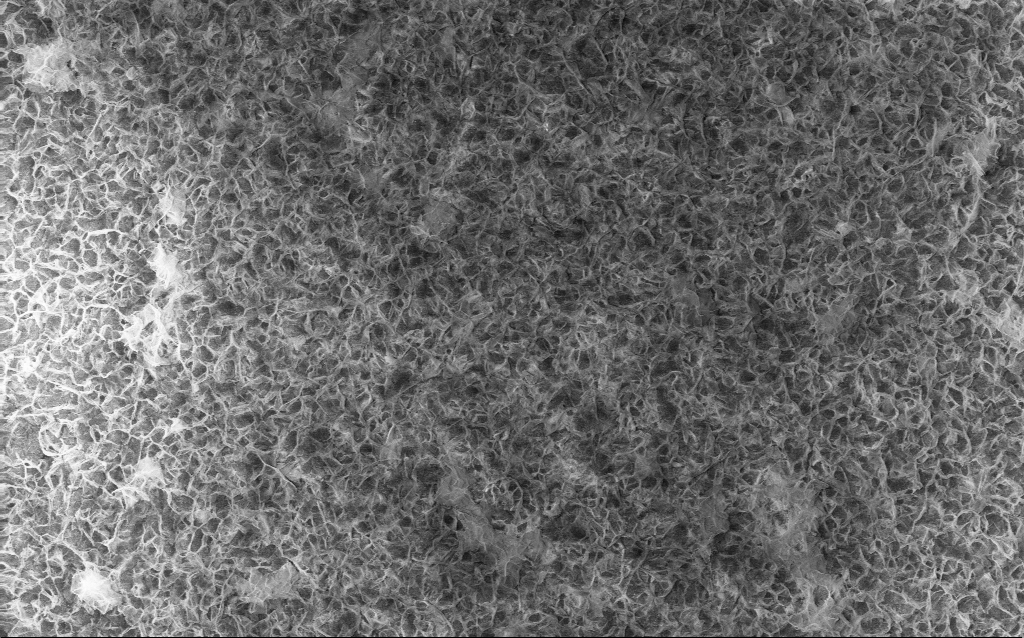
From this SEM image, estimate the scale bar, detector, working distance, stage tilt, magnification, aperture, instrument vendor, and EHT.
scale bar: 10000 nm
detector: InLens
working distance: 2.8 mm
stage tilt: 0°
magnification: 5 K X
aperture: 30 µm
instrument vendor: Zeiss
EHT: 10 kV